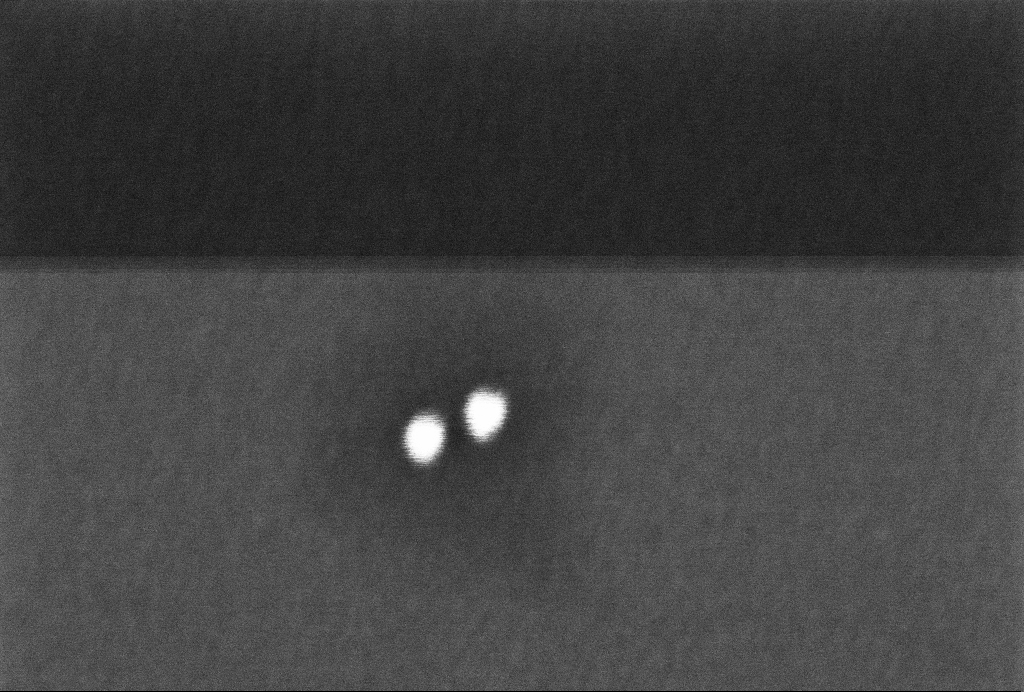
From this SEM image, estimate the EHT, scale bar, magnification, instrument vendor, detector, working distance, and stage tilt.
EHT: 2 kV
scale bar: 100 nm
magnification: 523.43 K X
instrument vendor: Zeiss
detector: InLens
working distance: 3.3 mm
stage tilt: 0°